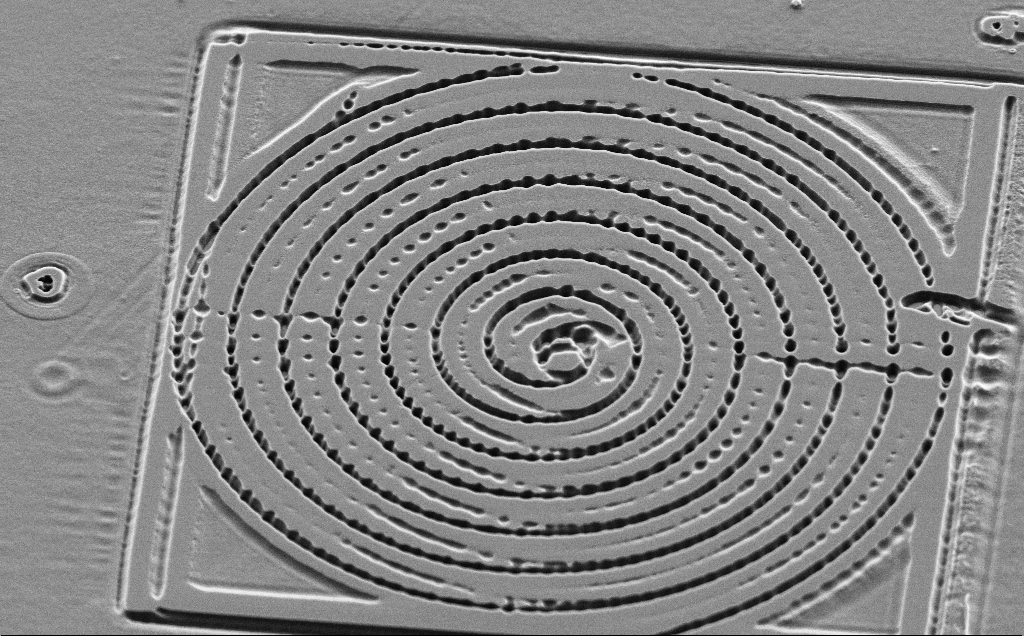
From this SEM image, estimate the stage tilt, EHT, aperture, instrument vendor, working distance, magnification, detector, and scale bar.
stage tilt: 45°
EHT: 5 kV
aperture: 30 µm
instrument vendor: Zeiss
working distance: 10 mm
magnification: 2.94 K X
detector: SE2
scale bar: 20000 nm